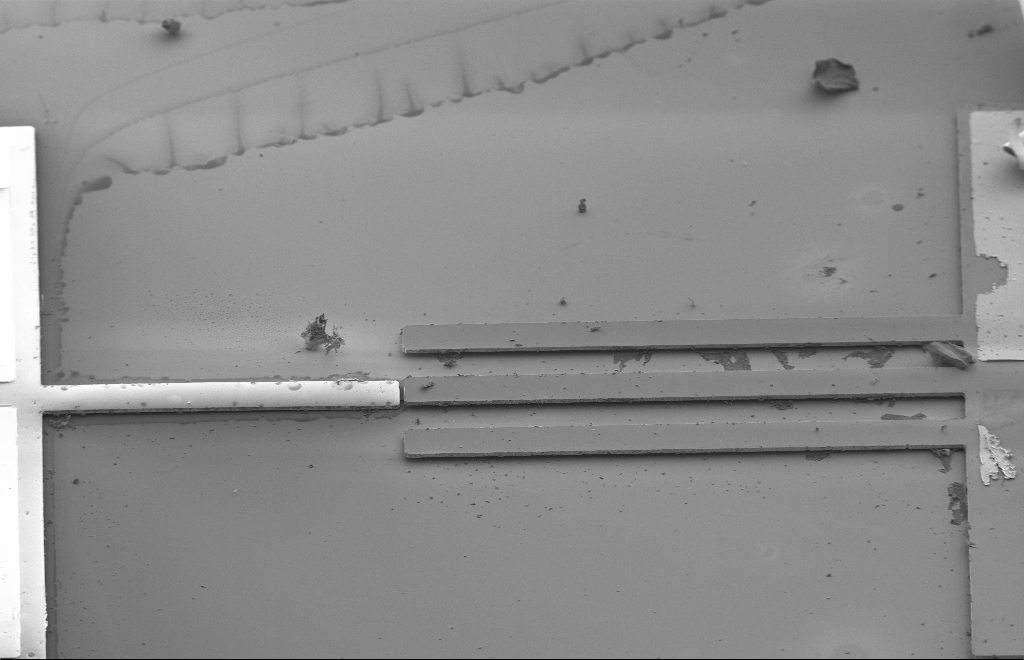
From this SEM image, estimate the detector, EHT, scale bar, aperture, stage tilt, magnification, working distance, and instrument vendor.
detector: SE2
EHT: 2 kV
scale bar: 20000 nm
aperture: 30 µm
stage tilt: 47.2°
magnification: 0.737 K X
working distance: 13 mm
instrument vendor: Zeiss